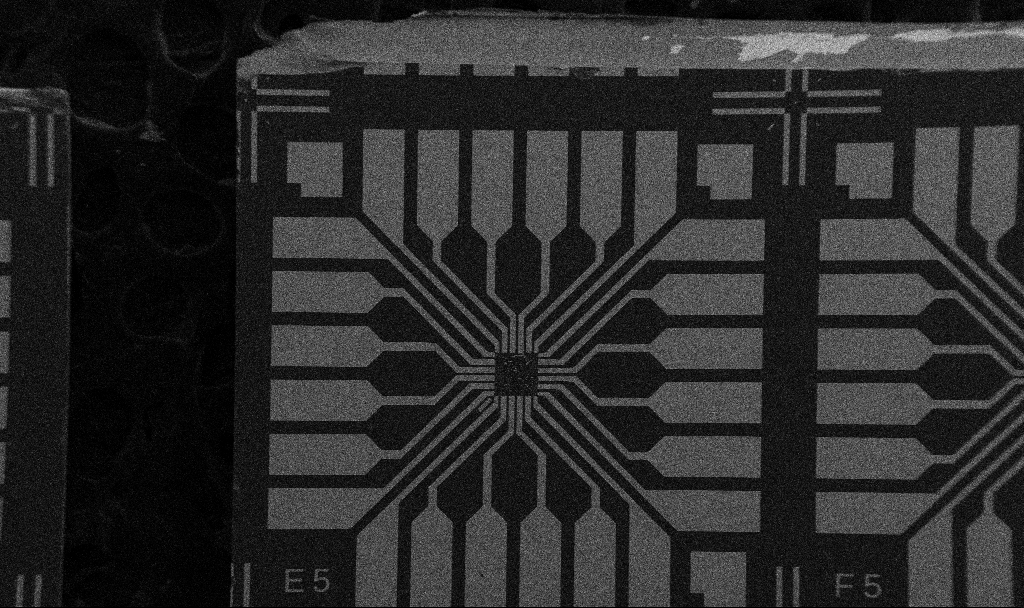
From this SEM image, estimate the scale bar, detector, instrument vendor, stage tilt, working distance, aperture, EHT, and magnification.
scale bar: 200000 nm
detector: SE2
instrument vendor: Zeiss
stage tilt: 0°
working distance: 10.7 mm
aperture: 30 µm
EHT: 5 kV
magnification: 0.1 K X